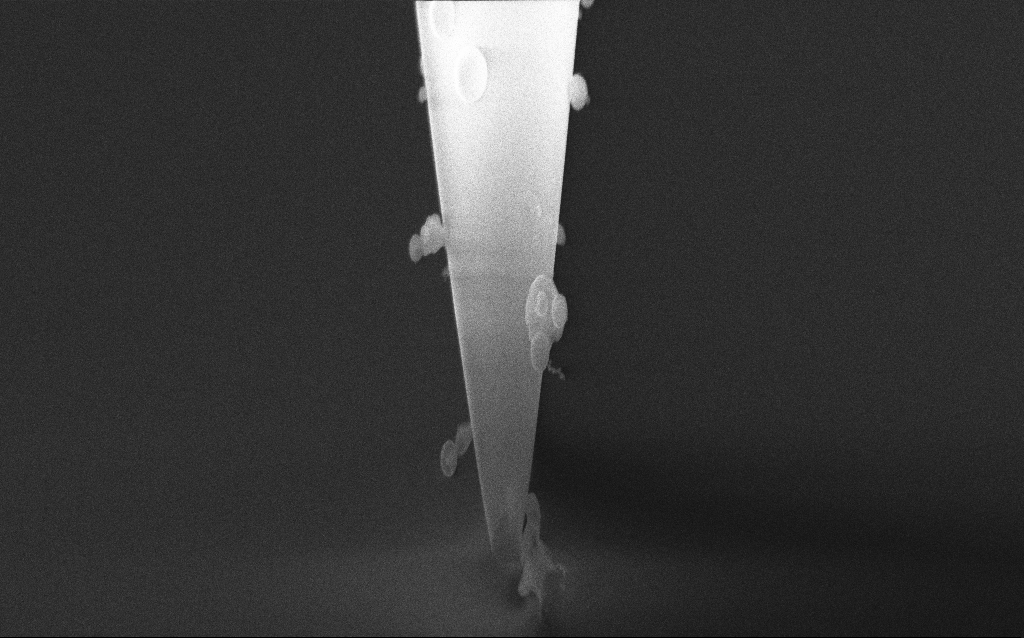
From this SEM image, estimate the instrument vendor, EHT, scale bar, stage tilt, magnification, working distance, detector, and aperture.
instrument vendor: Zeiss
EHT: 5 kV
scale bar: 1000 nm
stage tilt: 45°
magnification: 30.67 K X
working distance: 4 mm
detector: InLens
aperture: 30 µm